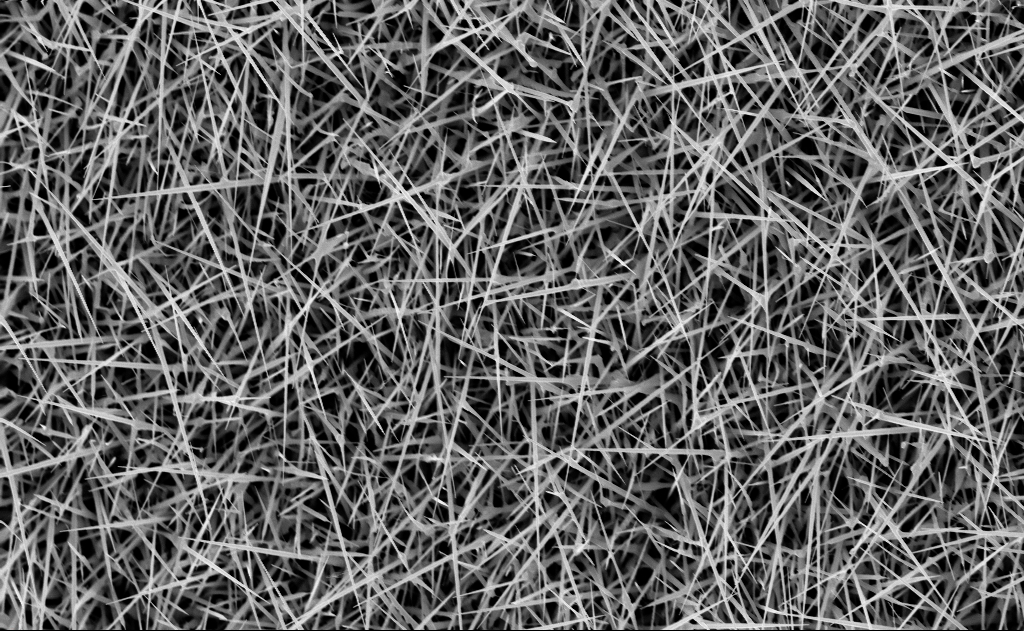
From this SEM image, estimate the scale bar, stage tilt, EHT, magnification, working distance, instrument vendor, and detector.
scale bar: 2000 nm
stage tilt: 0°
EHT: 10 kV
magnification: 10 K X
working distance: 10 mm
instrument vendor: Zeiss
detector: InLens